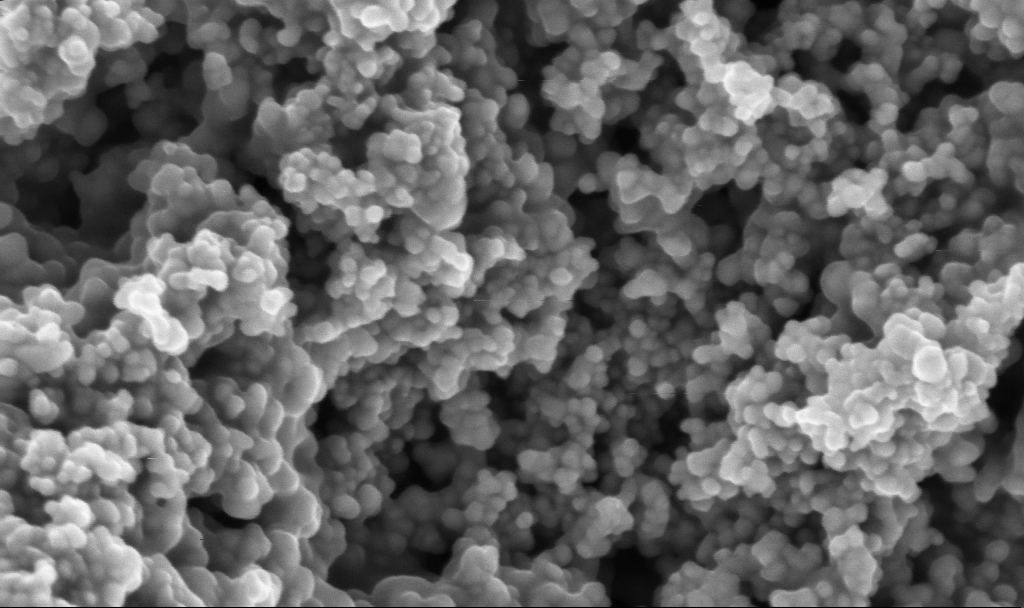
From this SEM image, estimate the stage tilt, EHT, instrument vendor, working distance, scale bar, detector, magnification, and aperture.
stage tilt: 0°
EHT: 3 kV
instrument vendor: Zeiss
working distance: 2.4 mm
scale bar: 100 nm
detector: InLens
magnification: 200 K X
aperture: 30 µm